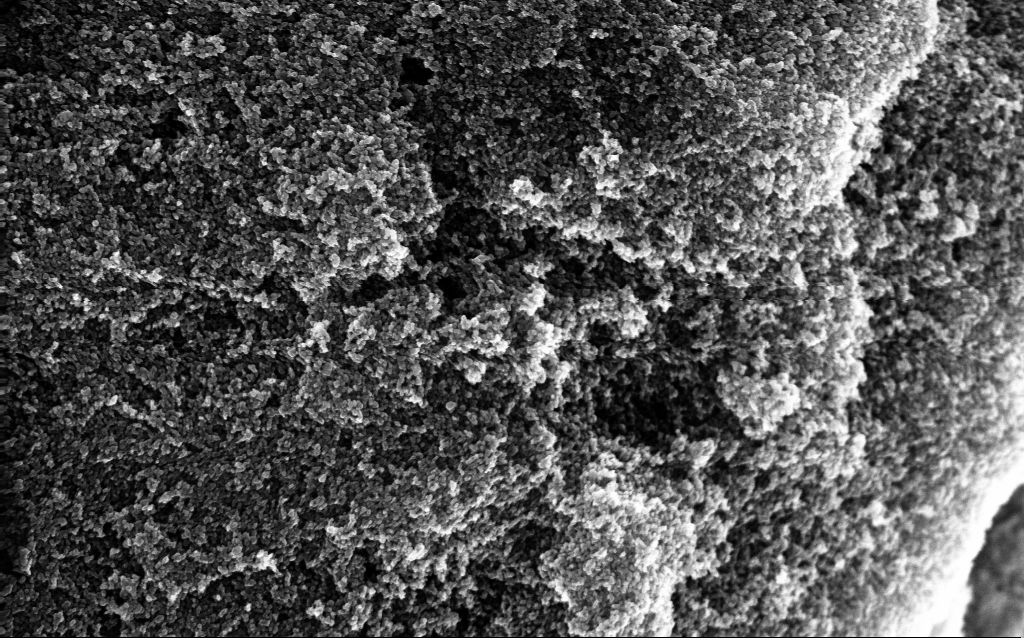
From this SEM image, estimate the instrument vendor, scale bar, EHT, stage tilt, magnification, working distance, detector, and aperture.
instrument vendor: Zeiss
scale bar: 1000 nm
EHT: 10 kV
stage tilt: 0°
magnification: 65.04 K X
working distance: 2.8 mm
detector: InLens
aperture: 30 µm